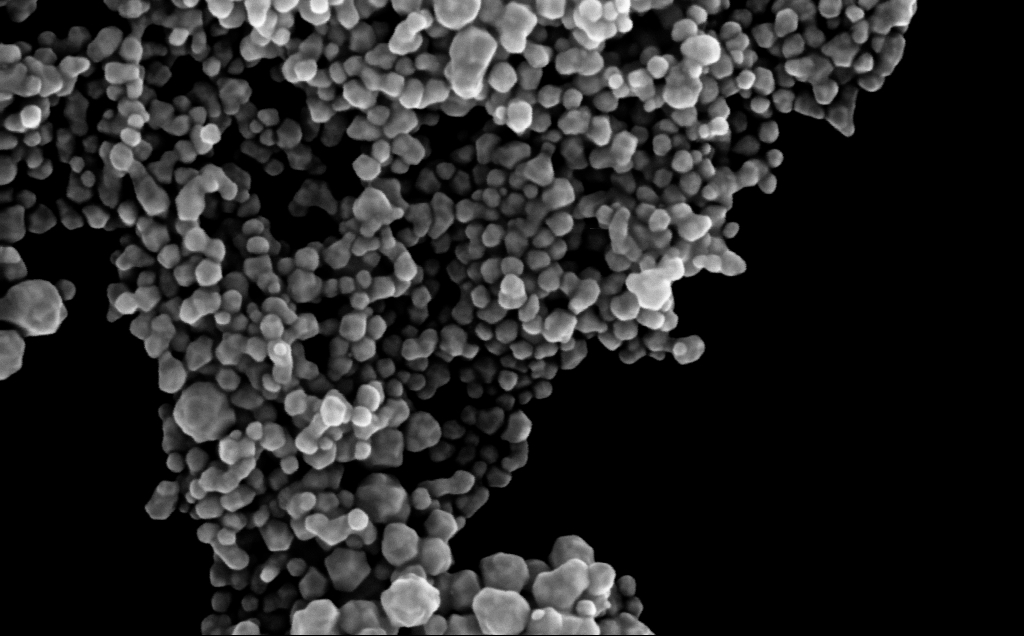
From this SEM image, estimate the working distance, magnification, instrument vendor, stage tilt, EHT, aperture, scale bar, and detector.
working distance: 3 mm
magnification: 226.69 K X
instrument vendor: Zeiss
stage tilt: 0°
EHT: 10 kV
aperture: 30 µm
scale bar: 200 nm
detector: InLens